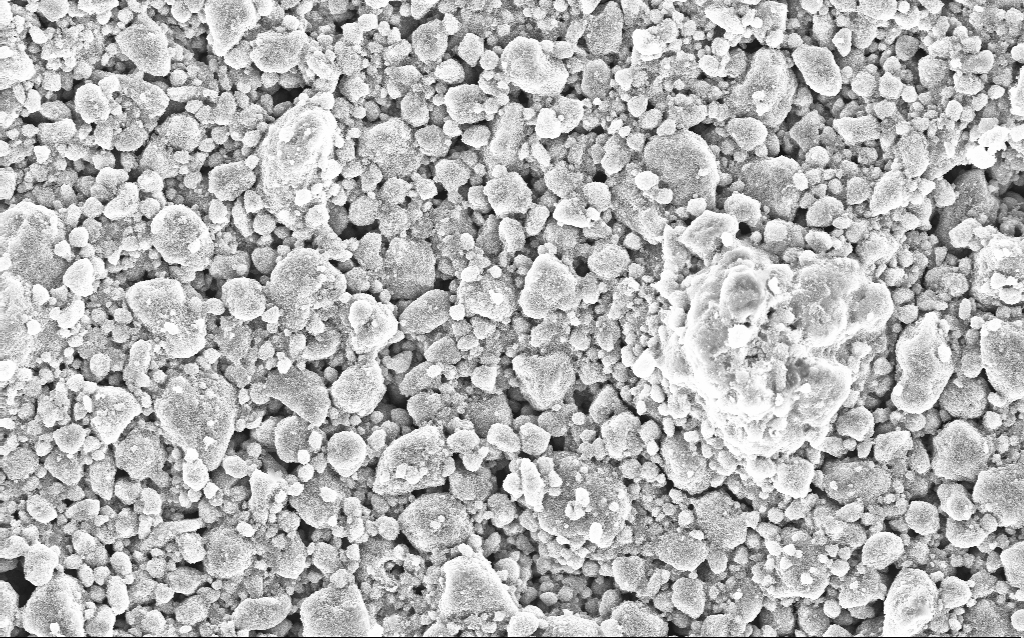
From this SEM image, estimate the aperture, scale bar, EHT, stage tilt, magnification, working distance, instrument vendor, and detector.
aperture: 30 µm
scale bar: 10000 nm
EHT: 10 kV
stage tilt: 0°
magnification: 3.59 K X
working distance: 3.8 mm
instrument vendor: Zeiss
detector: InLens